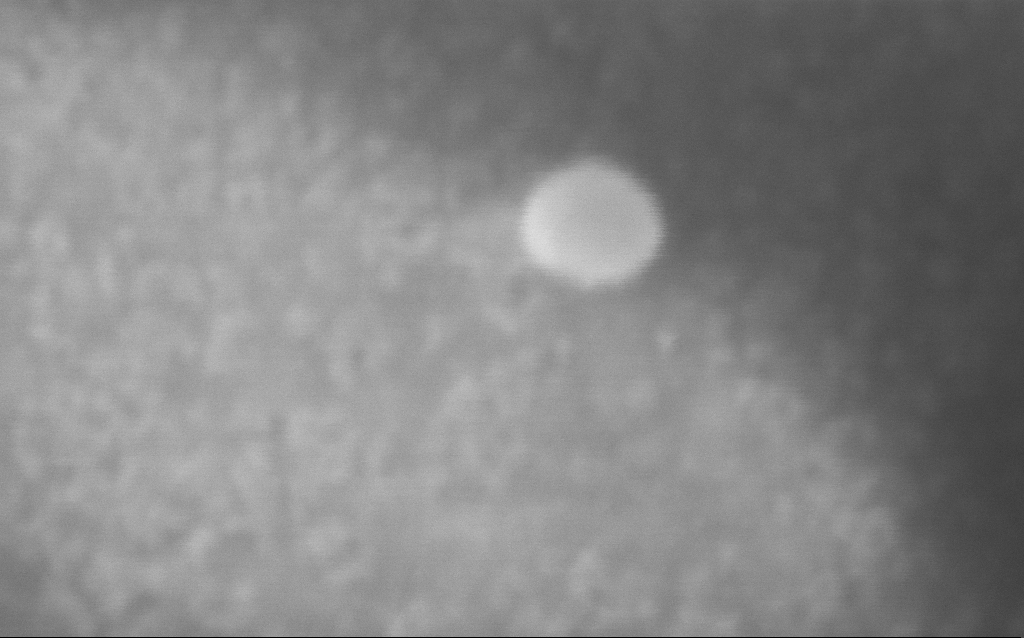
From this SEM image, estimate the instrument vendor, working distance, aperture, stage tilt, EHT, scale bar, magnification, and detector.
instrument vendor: Zeiss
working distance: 2 mm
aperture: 30 µm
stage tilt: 0°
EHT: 10 kV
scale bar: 20 nm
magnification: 1670.3 K X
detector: InLens